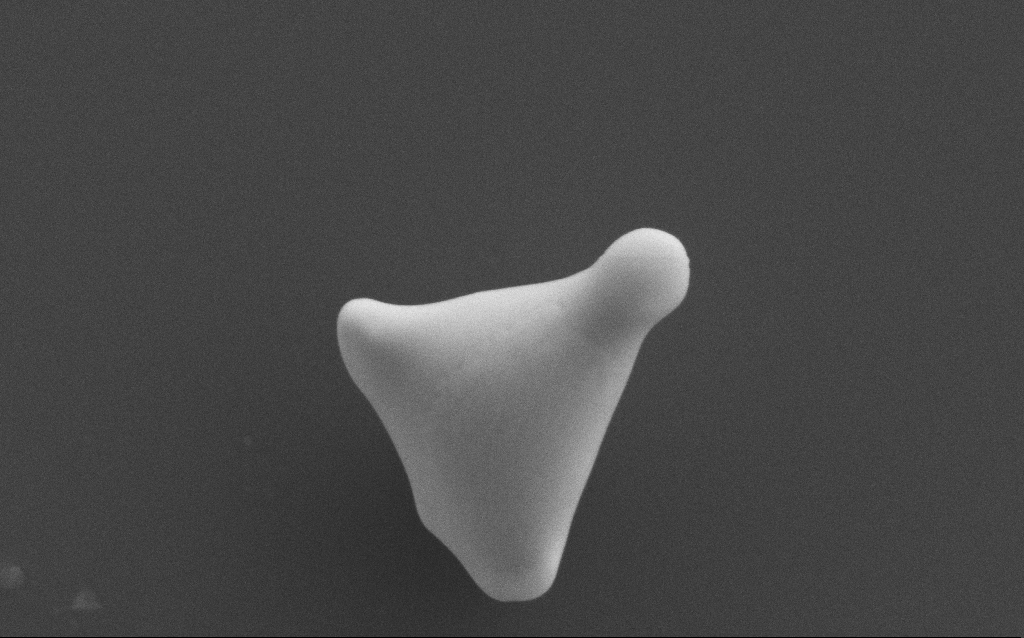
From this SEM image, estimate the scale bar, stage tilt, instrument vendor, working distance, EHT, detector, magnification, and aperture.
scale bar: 1000 nm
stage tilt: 0°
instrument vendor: Zeiss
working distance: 4 mm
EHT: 10 kV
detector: SE2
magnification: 46.26 K X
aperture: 30 µm